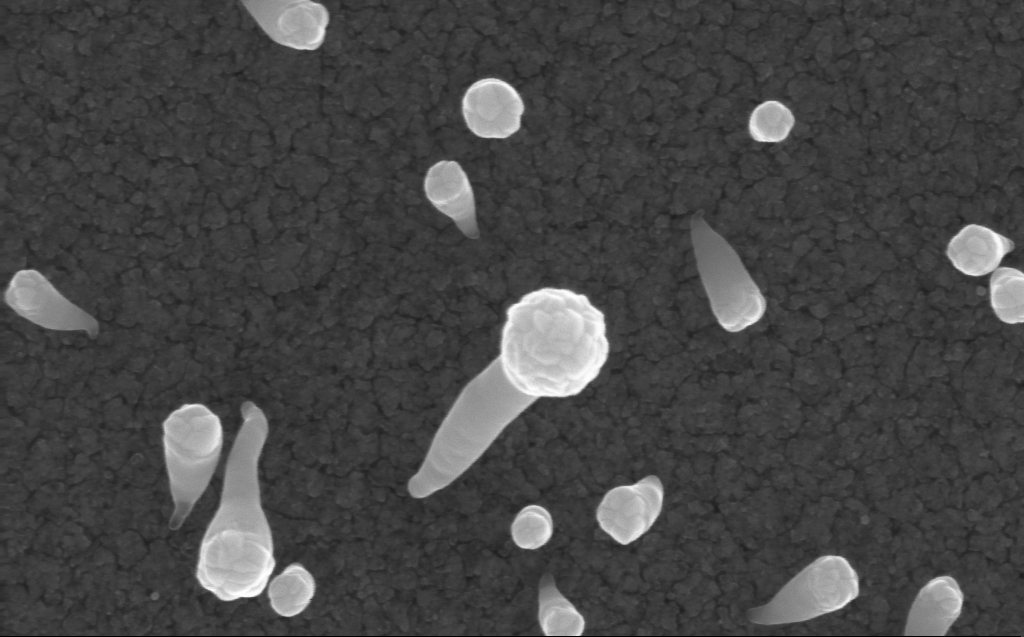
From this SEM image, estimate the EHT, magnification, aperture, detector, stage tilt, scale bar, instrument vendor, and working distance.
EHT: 10 kV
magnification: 152.22 K X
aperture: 30 µm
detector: InLens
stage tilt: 0°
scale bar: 200 nm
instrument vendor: Zeiss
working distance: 3 mm